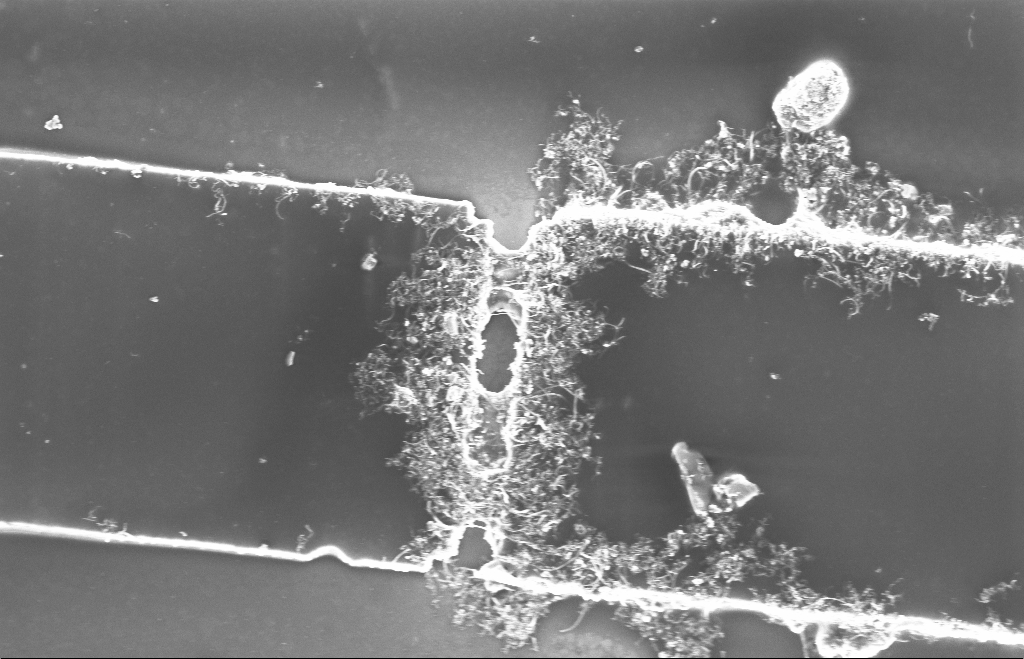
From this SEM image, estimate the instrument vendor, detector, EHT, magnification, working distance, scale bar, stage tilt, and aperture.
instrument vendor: Zeiss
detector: InLens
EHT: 5 kV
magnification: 7.49 K X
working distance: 11 mm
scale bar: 2000 nm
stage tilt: -1.6°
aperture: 20 µm